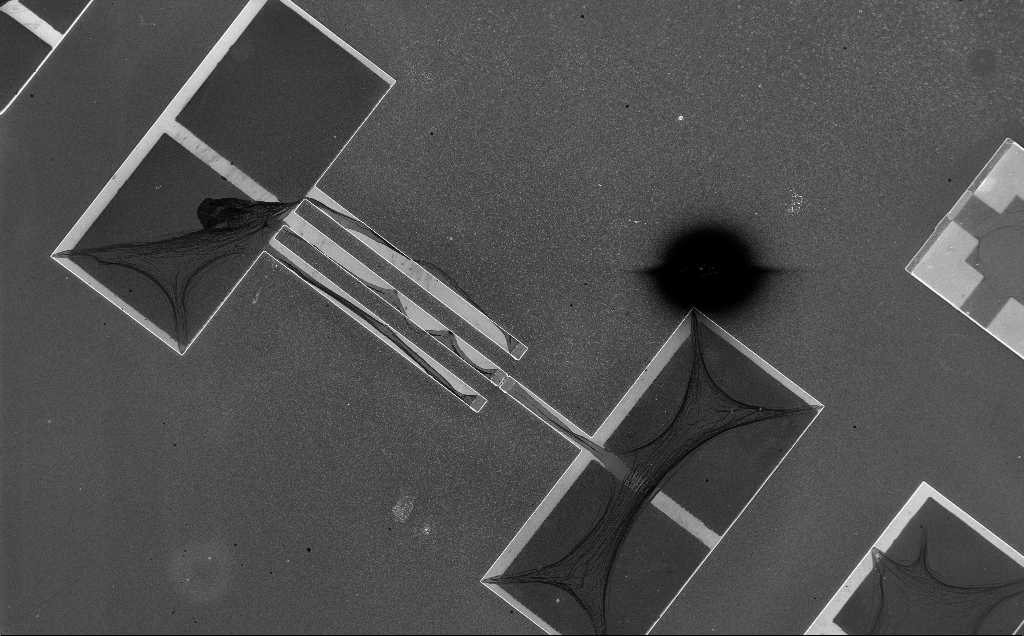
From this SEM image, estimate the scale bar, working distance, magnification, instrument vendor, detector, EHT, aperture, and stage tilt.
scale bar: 100000 nm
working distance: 10 mm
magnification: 0.305 K X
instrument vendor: Zeiss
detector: InLens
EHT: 5 kV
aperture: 30 µm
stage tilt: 0°